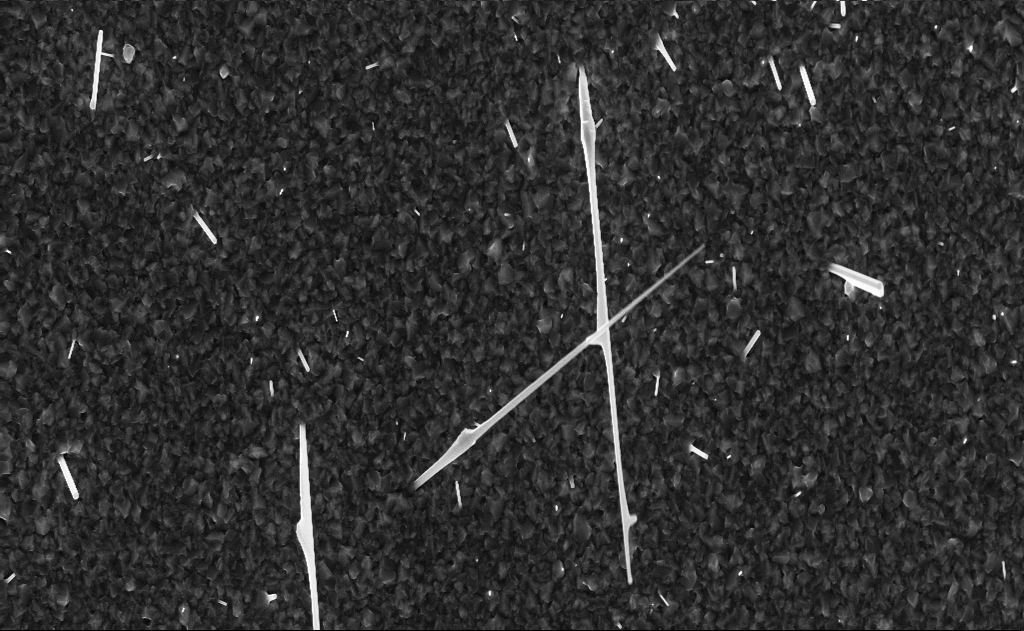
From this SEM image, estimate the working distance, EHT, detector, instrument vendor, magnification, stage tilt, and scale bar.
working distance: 6 mm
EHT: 10 kV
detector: InLens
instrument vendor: Zeiss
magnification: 10 K X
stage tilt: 0°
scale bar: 2000 nm